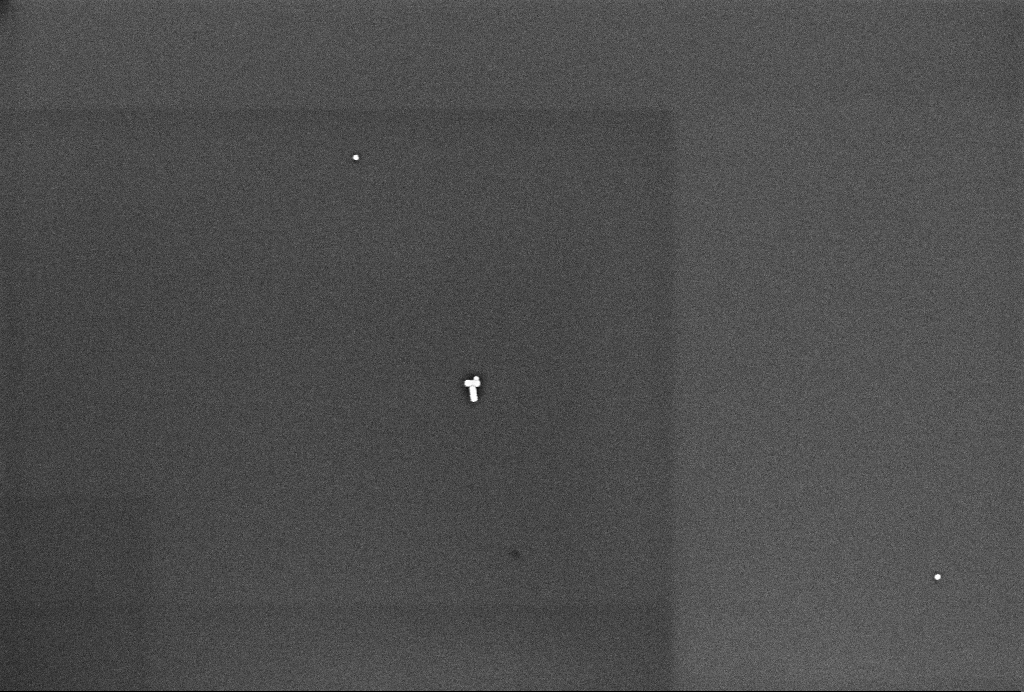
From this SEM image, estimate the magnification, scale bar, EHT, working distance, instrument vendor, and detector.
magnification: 80.44 K X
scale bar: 200 nm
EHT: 2 kV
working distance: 3.3 mm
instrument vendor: Zeiss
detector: InLens